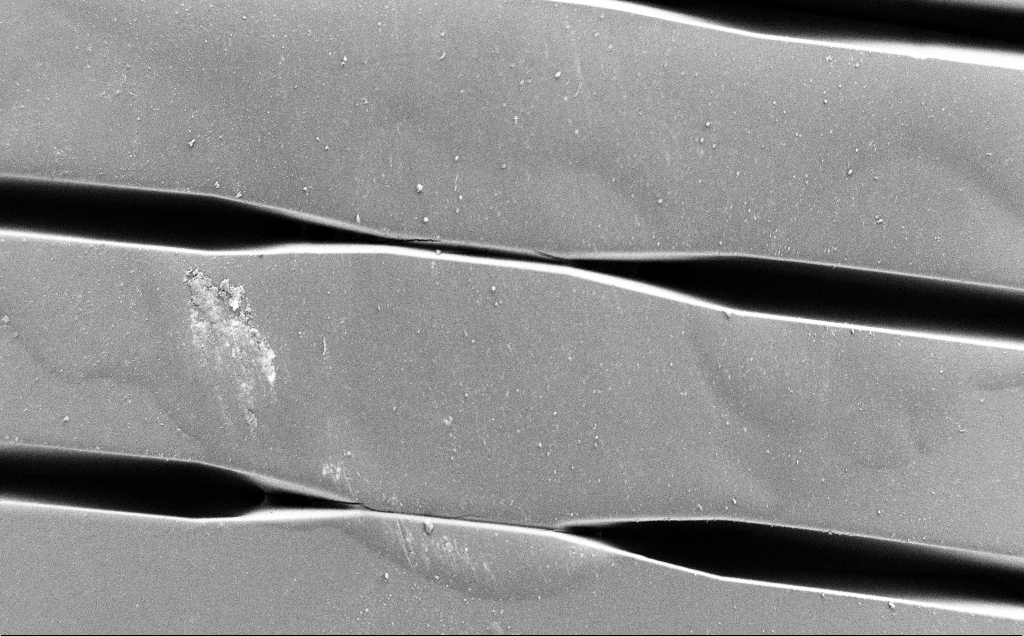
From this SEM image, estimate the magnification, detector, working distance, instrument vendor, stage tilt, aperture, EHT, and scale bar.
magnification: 0.667 K X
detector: SE2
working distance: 5 mm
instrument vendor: Zeiss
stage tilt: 0°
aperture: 120 µm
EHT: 1.2 kV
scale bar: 100000 nm